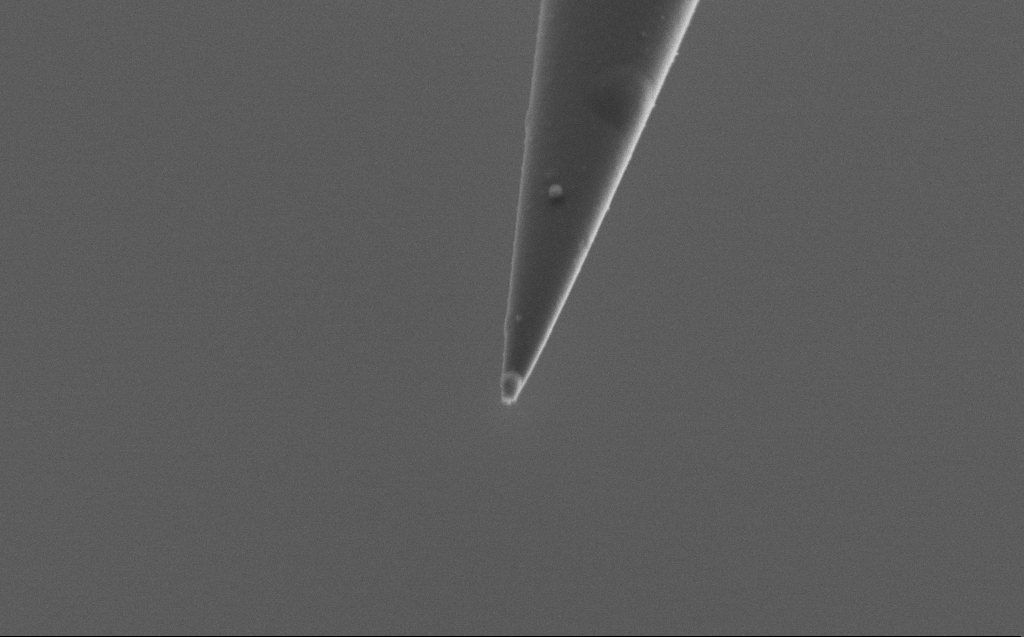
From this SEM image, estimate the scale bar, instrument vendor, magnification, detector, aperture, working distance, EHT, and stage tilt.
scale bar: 1000 nm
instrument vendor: Zeiss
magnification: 50 K X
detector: SE2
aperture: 30 µm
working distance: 5 mm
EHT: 2 kV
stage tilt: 45°